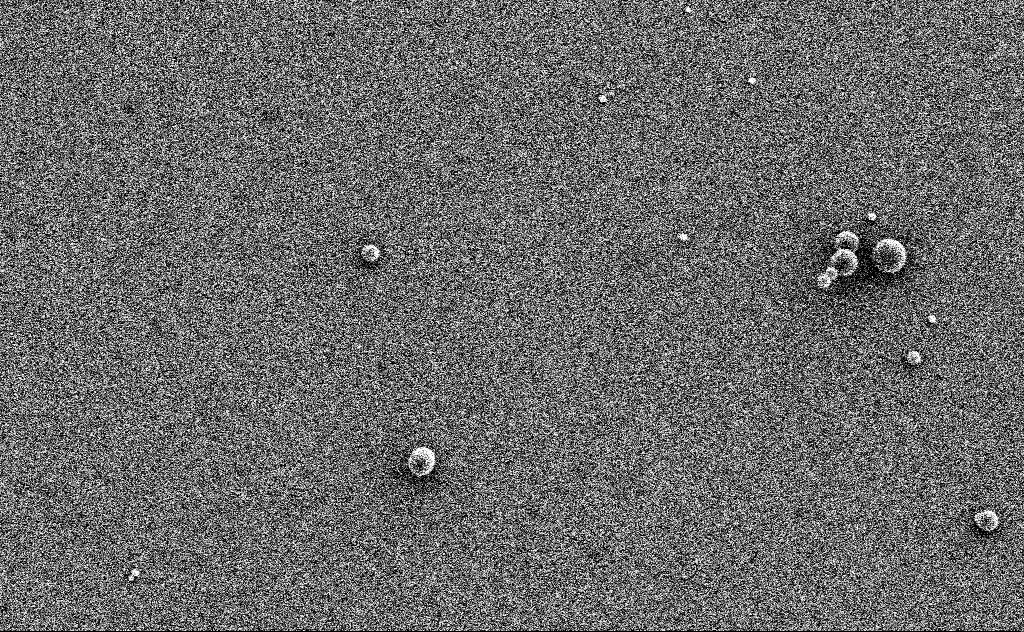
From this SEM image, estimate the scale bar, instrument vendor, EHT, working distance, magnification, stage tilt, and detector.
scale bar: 2000 nm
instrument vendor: Zeiss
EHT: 3 kV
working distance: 11 mm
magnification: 10.79 K X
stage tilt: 0°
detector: SE2